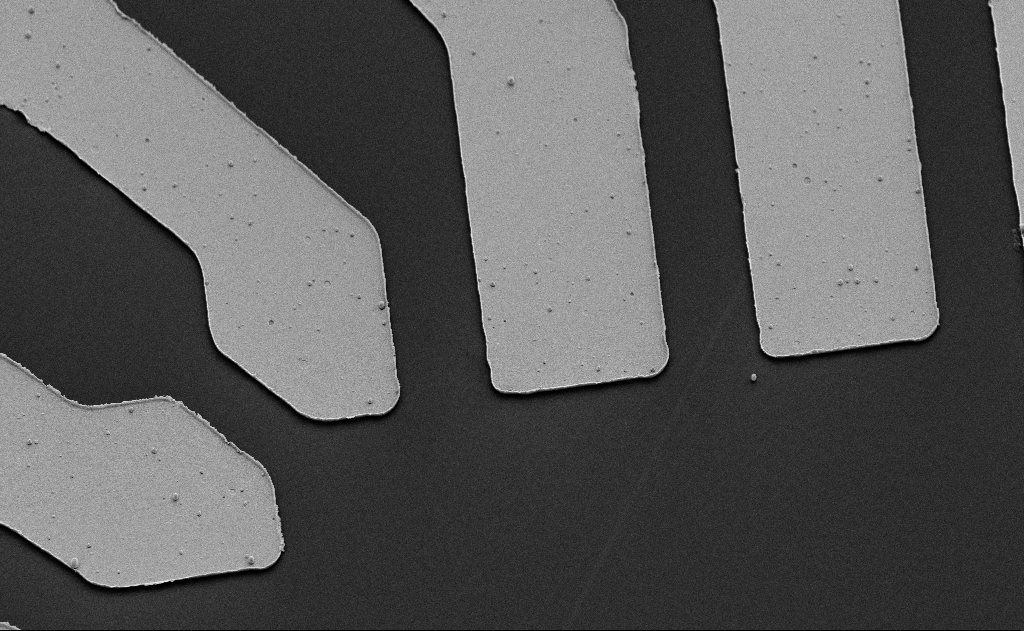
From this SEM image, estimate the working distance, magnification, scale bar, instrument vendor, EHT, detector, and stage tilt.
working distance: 10 mm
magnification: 3.34 K X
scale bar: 10000 nm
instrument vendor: Zeiss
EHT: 5 kV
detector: SE2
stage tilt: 45°